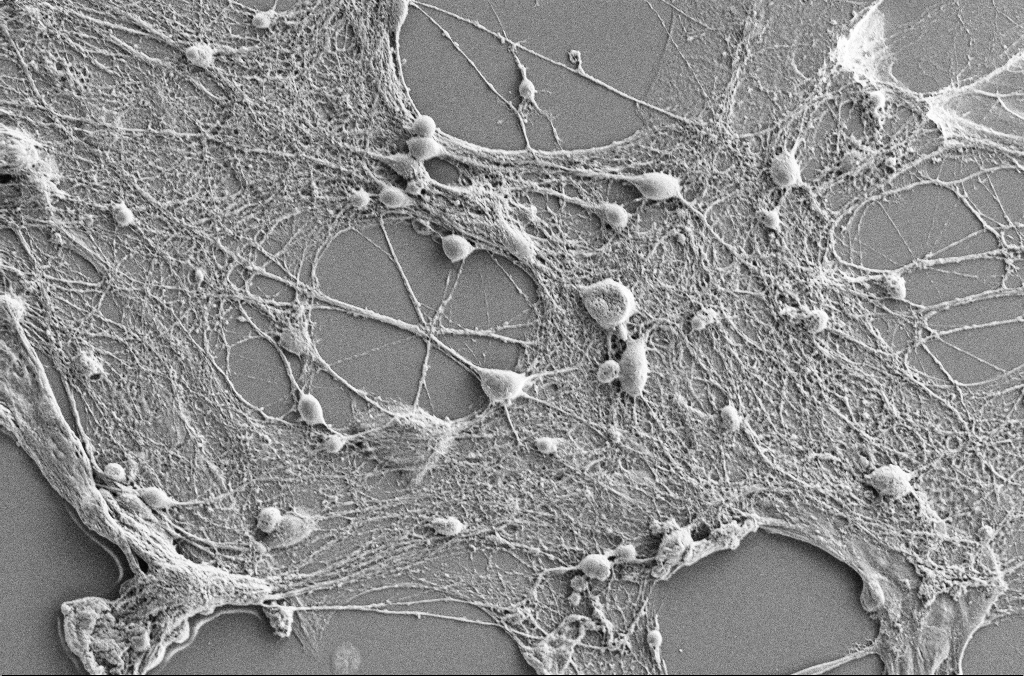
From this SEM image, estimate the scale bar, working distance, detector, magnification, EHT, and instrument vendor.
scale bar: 20000 nm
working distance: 3.7 mm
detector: SE2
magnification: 1 K X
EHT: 7 kV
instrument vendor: Zeiss